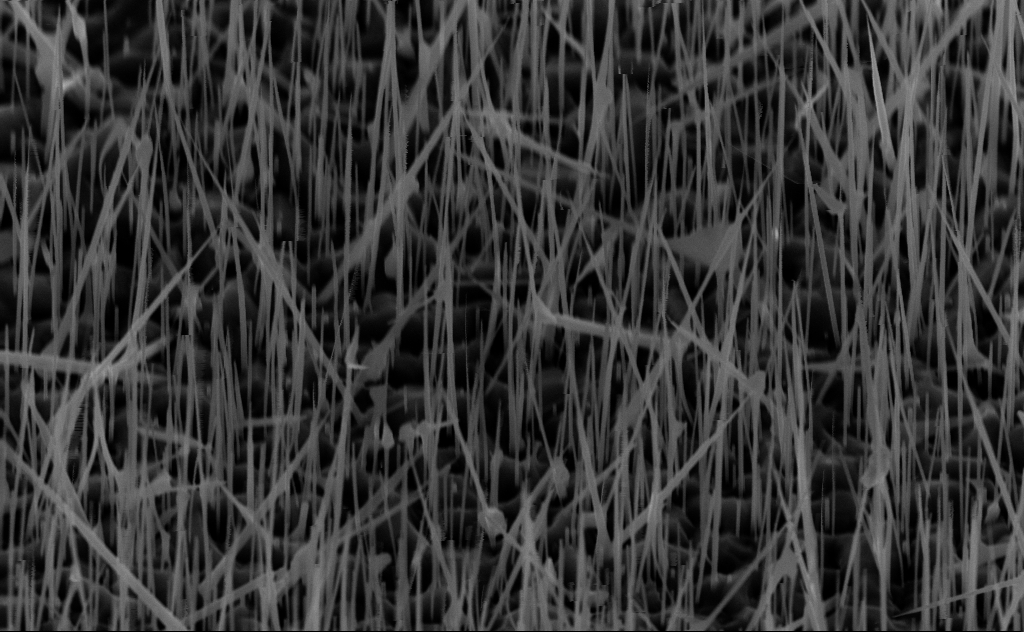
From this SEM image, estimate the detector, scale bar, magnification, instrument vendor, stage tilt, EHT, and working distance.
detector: InLens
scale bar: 1000 nm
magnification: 40 K X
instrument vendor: Zeiss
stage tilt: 44.2°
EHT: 10 kV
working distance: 6 mm